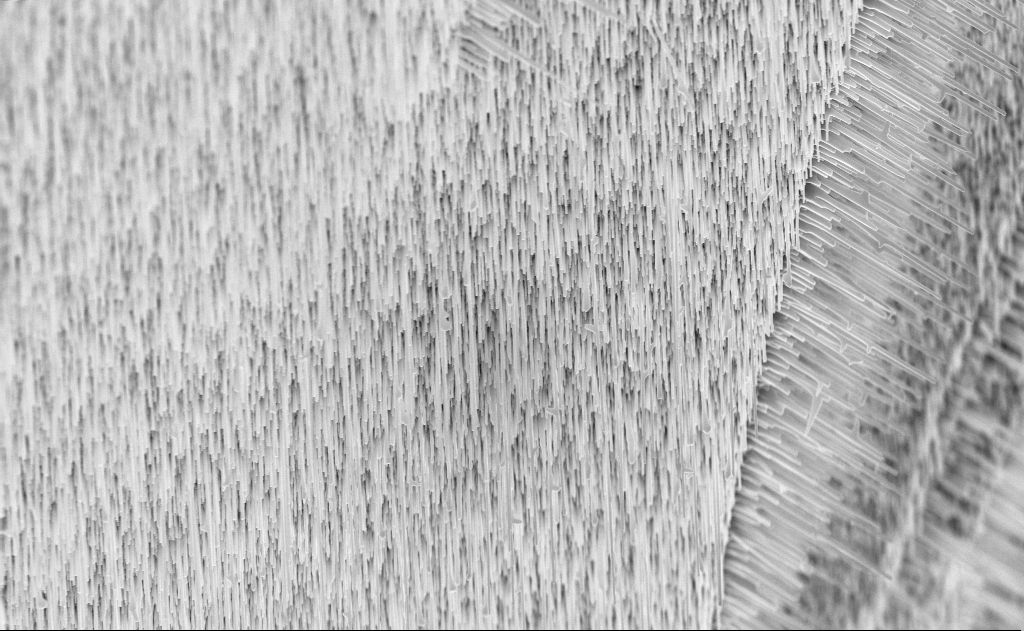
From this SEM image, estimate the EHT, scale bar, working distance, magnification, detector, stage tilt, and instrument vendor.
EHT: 10 kV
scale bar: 2000 nm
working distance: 6 mm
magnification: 10 K X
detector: InLens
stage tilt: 0°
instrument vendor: Zeiss